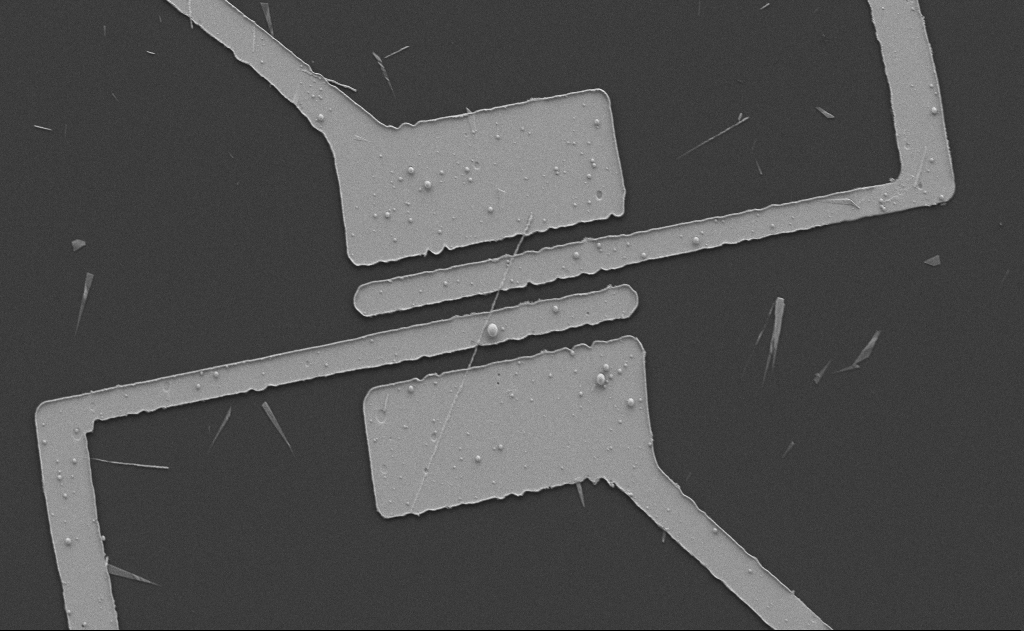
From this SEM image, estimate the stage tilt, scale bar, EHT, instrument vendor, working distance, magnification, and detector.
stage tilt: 0°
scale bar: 10000 nm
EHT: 5 kV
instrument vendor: Zeiss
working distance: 10 mm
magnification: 3.53 K X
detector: SE2